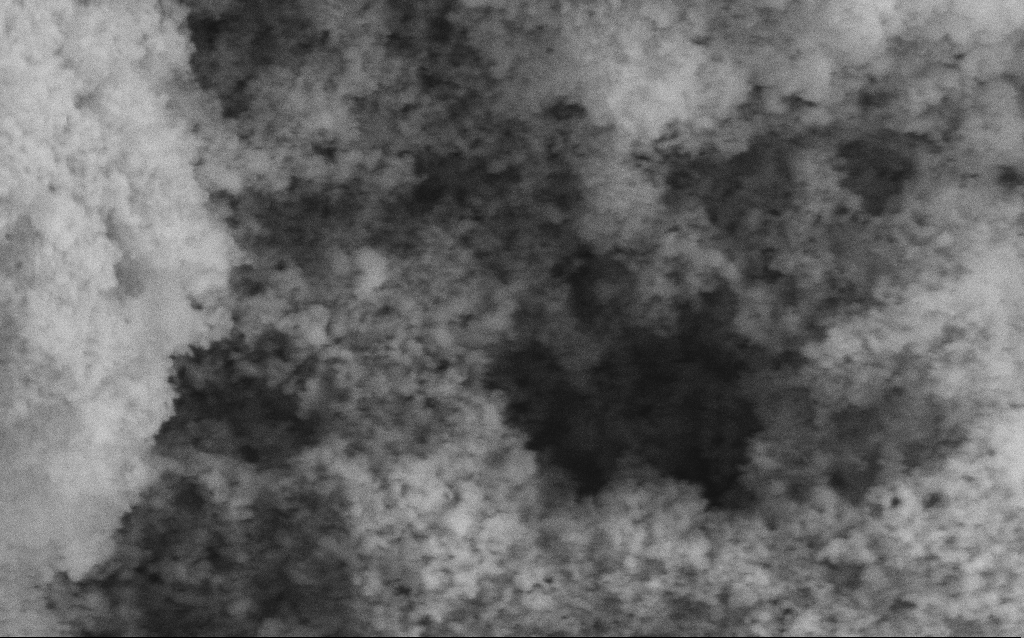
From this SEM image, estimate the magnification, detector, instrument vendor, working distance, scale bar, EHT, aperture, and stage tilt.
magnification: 114.64 K X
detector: SE2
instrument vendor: Zeiss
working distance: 4.2 mm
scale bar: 200 nm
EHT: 5 kV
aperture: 30 µm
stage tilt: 0°